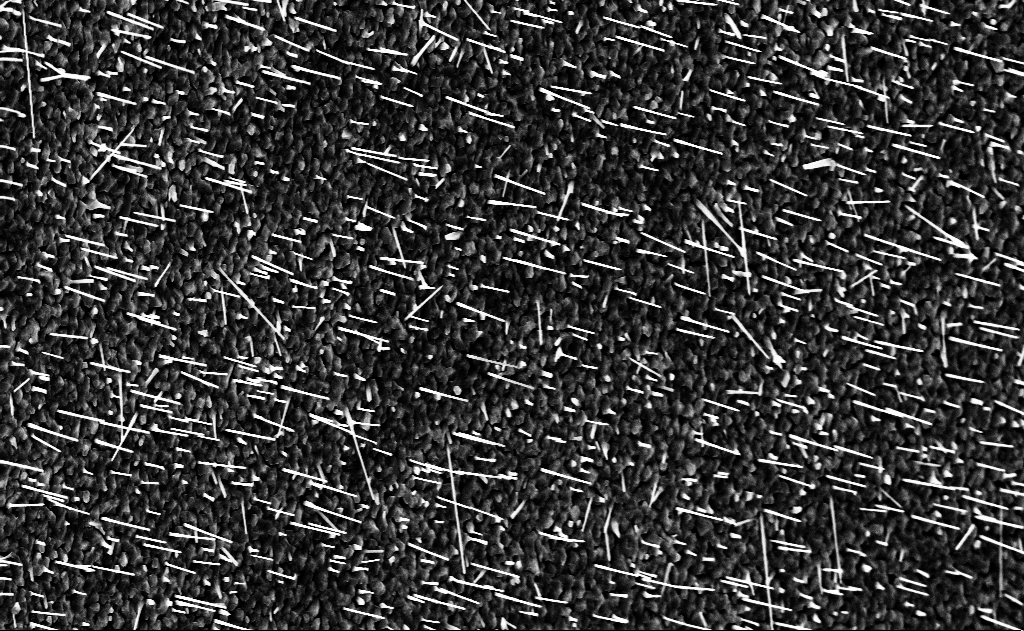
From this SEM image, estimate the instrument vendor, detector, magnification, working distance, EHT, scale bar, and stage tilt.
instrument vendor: Zeiss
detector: InLens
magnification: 10 K X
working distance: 14 mm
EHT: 10 kV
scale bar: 2000 nm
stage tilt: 0°